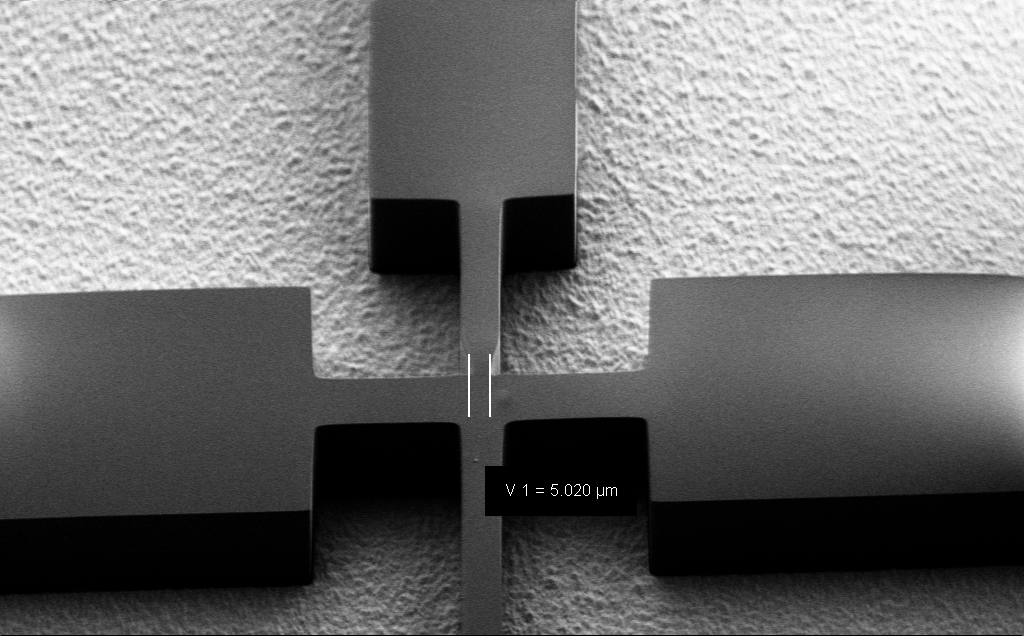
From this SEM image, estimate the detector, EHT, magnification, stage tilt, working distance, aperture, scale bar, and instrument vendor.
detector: SE2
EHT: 1.5 kV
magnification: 1.54 K X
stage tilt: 37.9°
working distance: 7 mm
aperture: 30 µm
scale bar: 20000 nm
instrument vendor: Zeiss